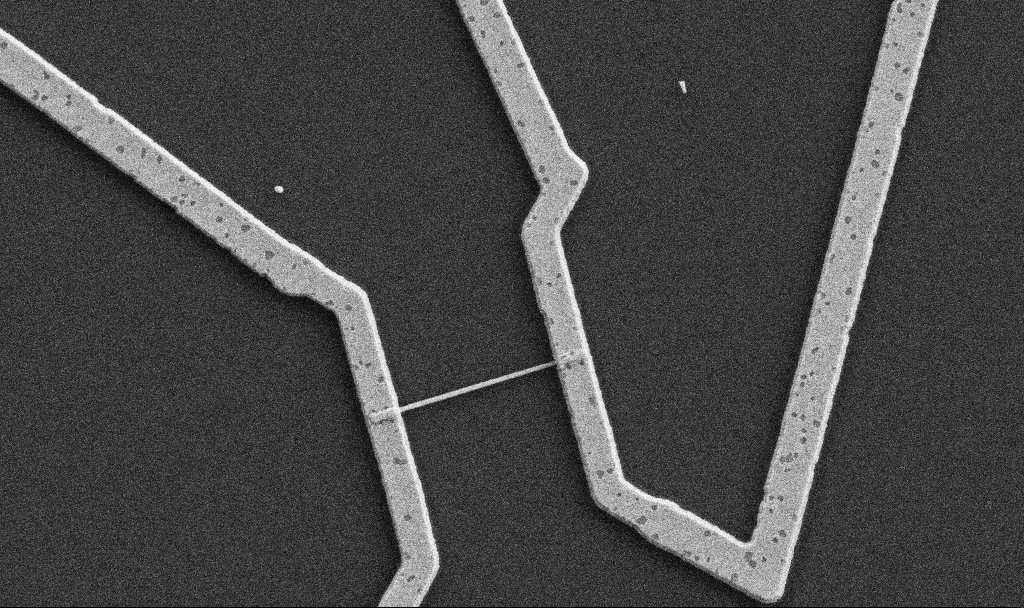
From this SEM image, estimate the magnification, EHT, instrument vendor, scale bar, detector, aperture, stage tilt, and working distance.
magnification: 20 K X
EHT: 5 kV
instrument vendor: Zeiss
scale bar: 1000 nm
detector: SE2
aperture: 30 µm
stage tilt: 0°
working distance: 10.7 mm